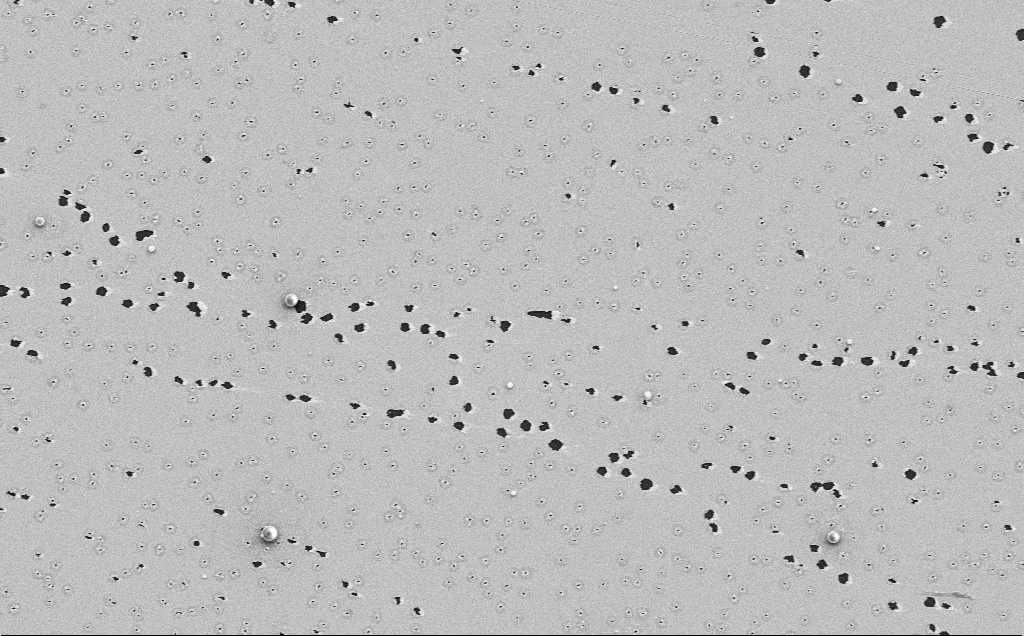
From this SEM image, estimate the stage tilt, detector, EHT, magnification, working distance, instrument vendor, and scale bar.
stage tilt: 0°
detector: SE2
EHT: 5 kV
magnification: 4.2 K X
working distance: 12 mm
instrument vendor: Zeiss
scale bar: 10000 nm